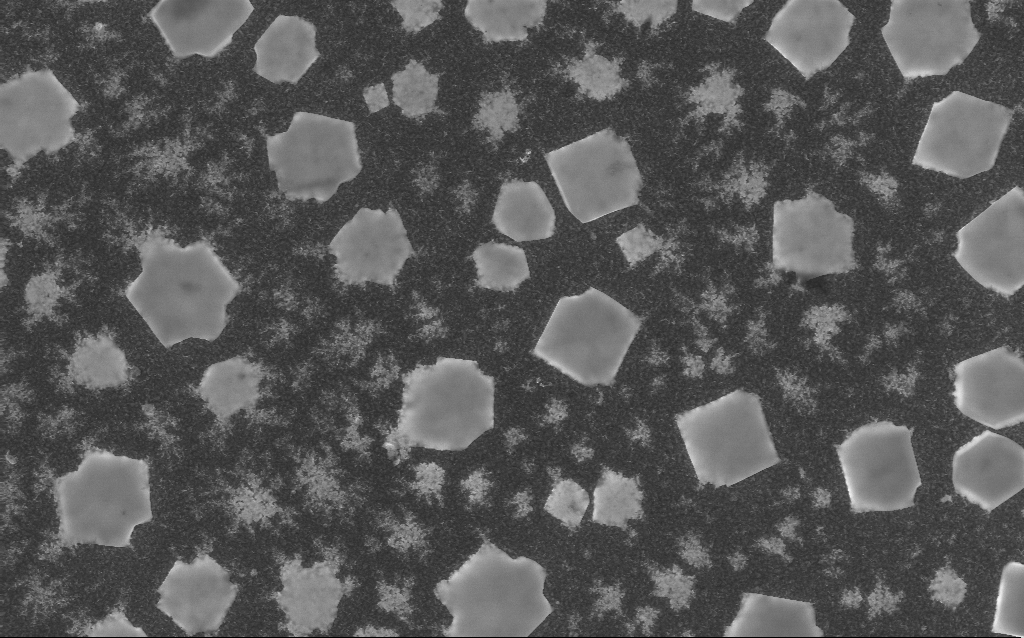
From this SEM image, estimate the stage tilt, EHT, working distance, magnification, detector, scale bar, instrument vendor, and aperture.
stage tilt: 0°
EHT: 10 kV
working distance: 4.5 mm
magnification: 5 K X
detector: InLens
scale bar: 10000 nm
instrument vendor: Zeiss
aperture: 30 µm